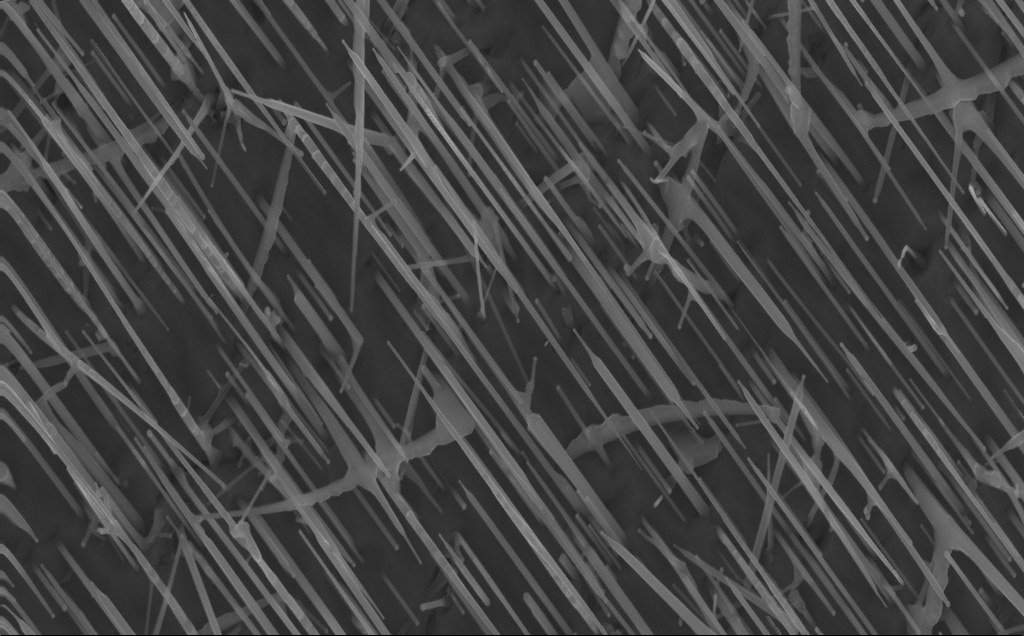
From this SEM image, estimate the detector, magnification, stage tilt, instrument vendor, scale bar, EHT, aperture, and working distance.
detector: InLens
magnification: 40 K X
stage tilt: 0°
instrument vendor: Zeiss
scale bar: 1000 nm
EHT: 10 kV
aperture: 30 µm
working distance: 4 mm